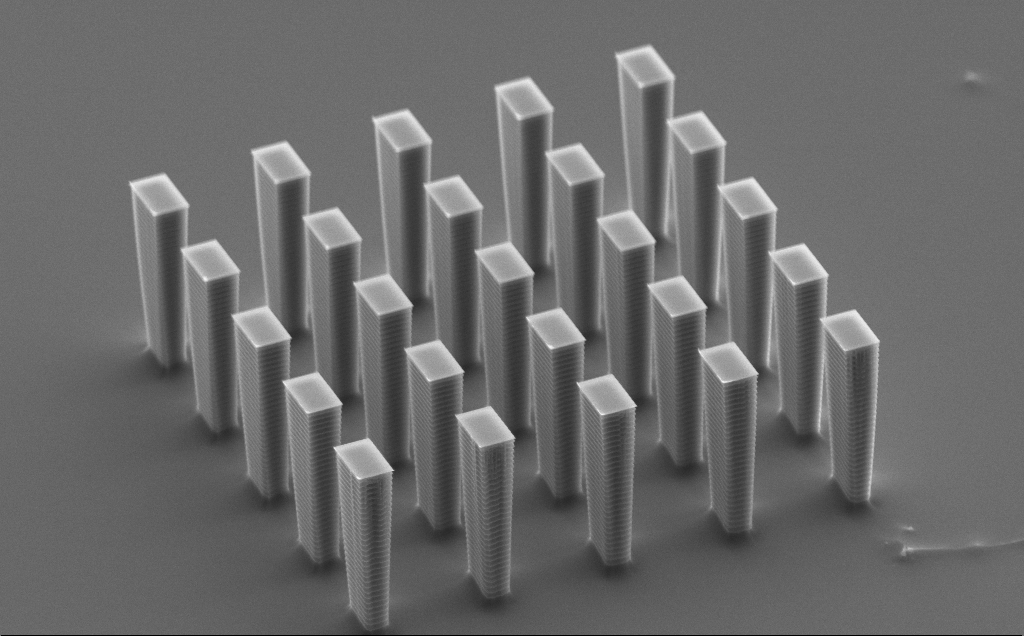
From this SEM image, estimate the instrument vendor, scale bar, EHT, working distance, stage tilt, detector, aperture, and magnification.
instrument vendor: Zeiss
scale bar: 10000 nm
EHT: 10 kV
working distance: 9 mm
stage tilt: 61.4°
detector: SE2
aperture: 30 µm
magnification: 4.87 K X